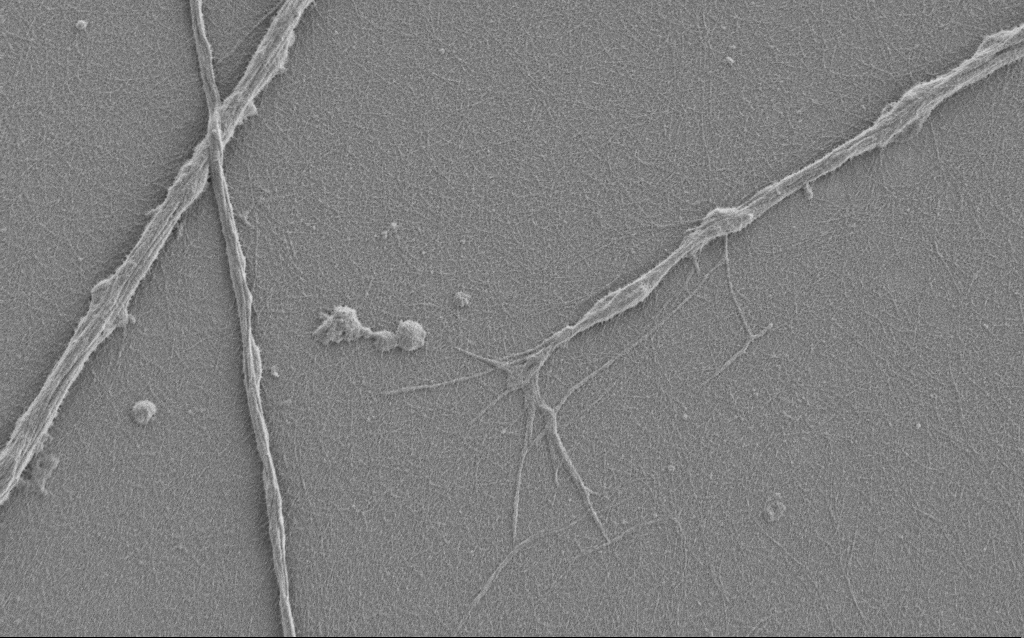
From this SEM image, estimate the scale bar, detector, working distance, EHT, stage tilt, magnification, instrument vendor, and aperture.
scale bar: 2000 nm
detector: SE2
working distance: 6 mm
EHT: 1 kV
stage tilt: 0°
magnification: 7.5 K X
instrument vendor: Zeiss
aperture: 30 µm